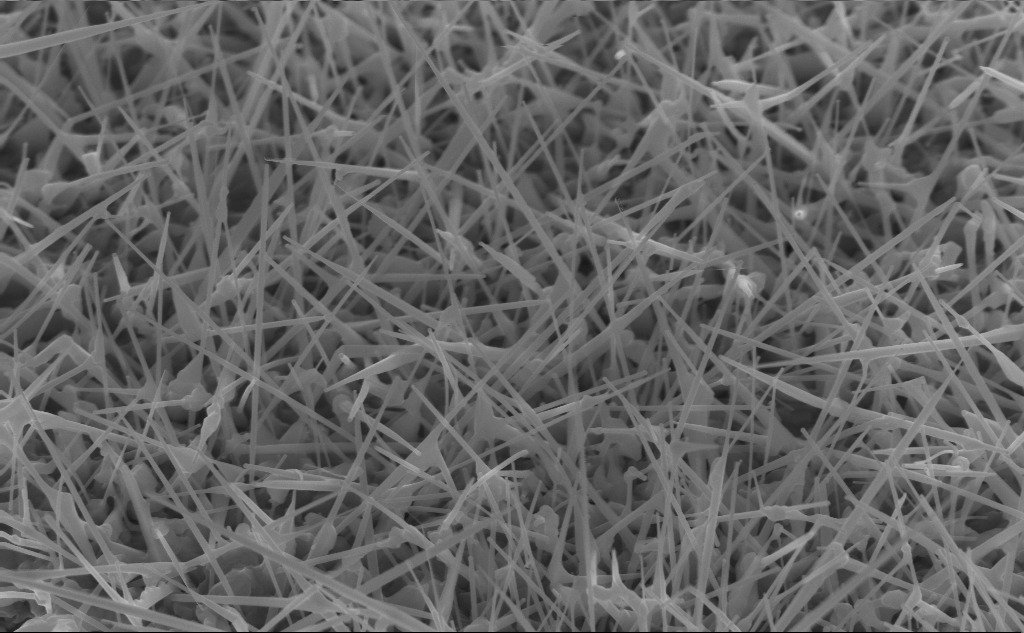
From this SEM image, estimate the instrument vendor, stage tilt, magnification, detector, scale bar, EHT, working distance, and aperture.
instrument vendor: Zeiss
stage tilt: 45°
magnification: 40 K X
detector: InLens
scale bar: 1000 nm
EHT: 10 kV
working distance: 4 mm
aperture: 30 µm